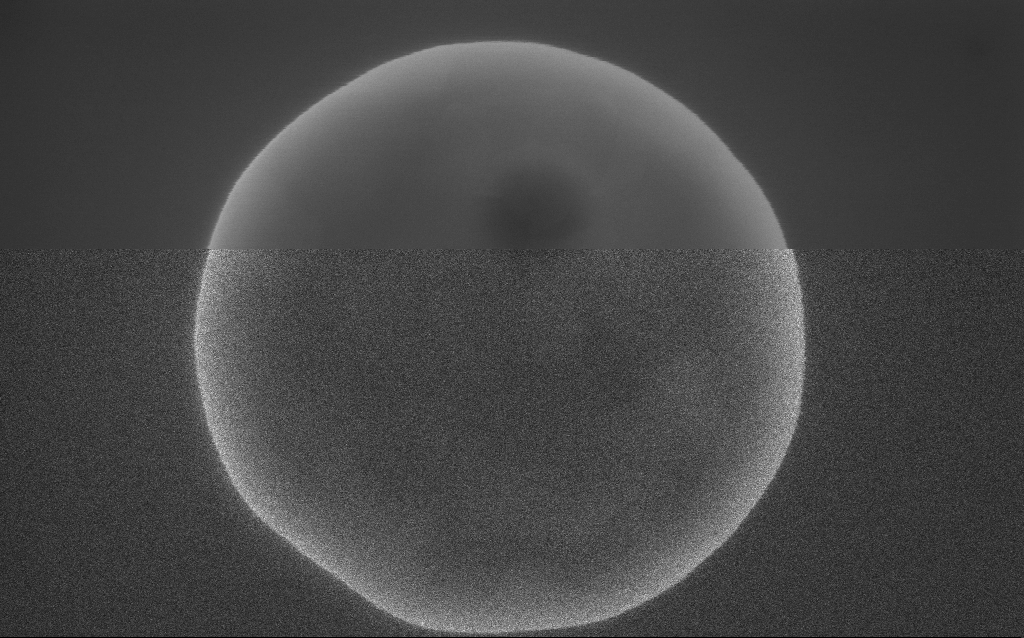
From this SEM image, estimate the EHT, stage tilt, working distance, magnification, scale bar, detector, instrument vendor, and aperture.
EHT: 10 kV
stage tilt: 0°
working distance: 3 mm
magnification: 175 K X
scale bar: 200 nm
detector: InLens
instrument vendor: Zeiss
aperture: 30 µm